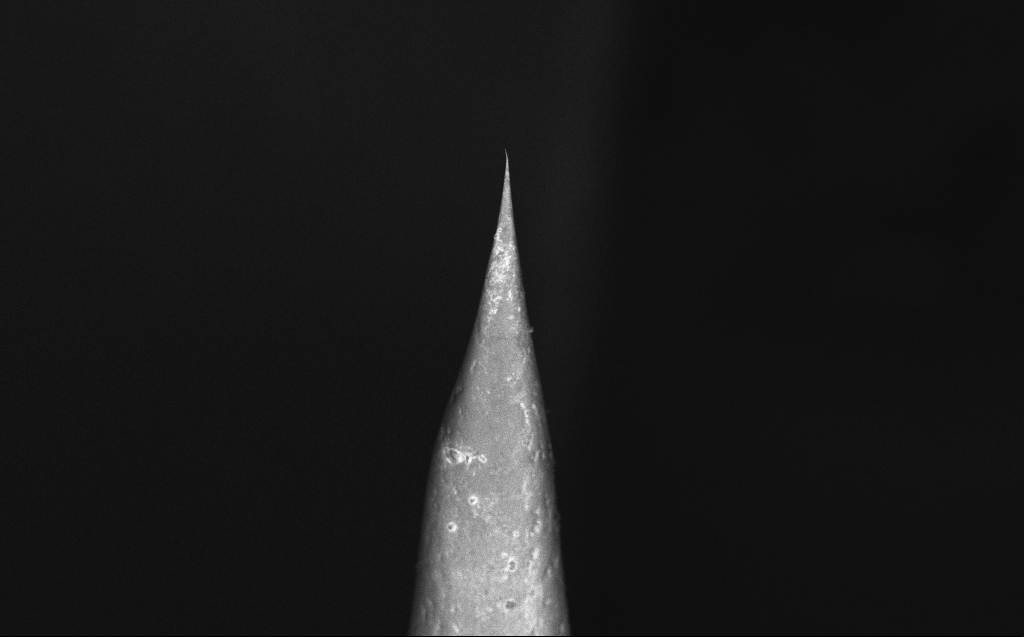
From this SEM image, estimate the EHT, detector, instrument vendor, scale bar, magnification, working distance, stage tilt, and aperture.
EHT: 10 kV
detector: InLens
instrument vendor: Zeiss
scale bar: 100000 nm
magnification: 0.547 K X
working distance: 6 mm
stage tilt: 40°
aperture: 30 µm